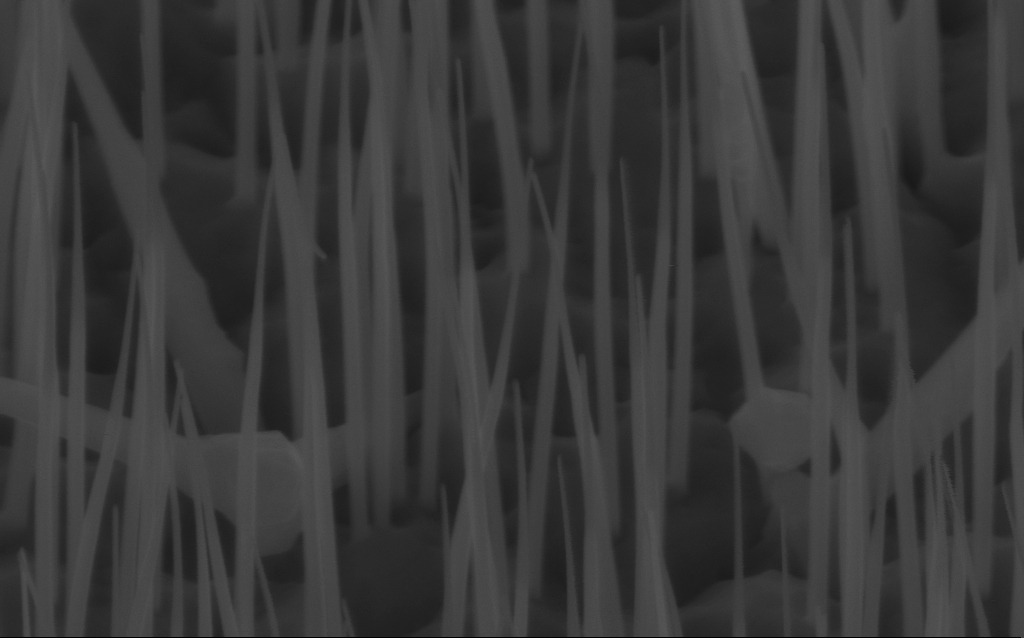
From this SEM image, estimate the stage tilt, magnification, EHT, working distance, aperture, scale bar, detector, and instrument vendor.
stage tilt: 45°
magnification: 100 K X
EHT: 10 kV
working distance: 6 mm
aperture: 30 µm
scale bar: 200 nm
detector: InLens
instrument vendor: Zeiss